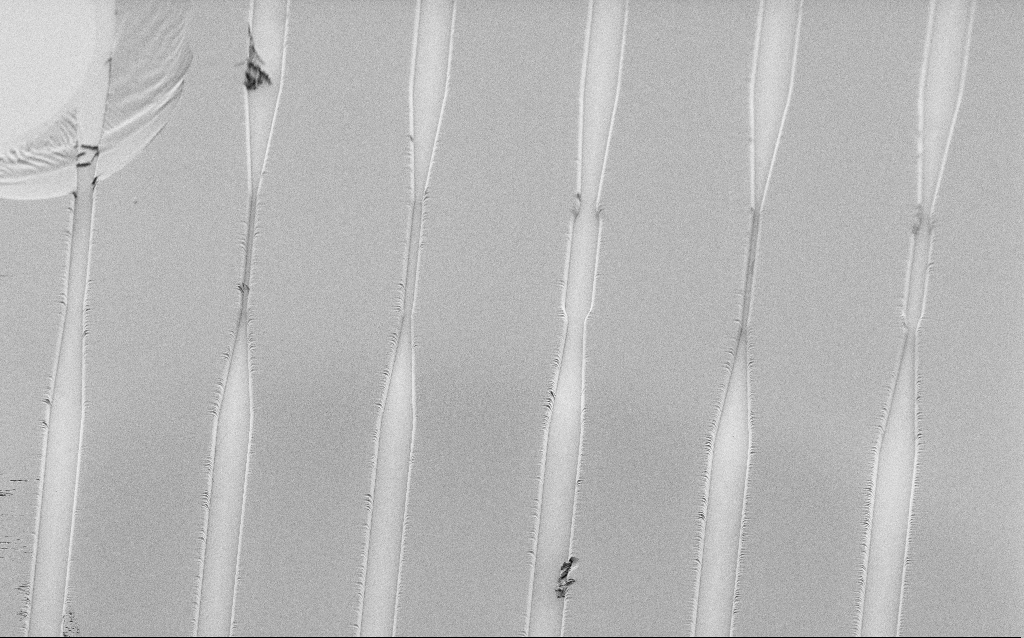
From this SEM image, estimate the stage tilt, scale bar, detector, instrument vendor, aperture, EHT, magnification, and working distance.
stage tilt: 45°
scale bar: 100000 nm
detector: SE2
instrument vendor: Zeiss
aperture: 30 µm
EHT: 1.2 kV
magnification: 0.572 K X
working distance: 8 mm